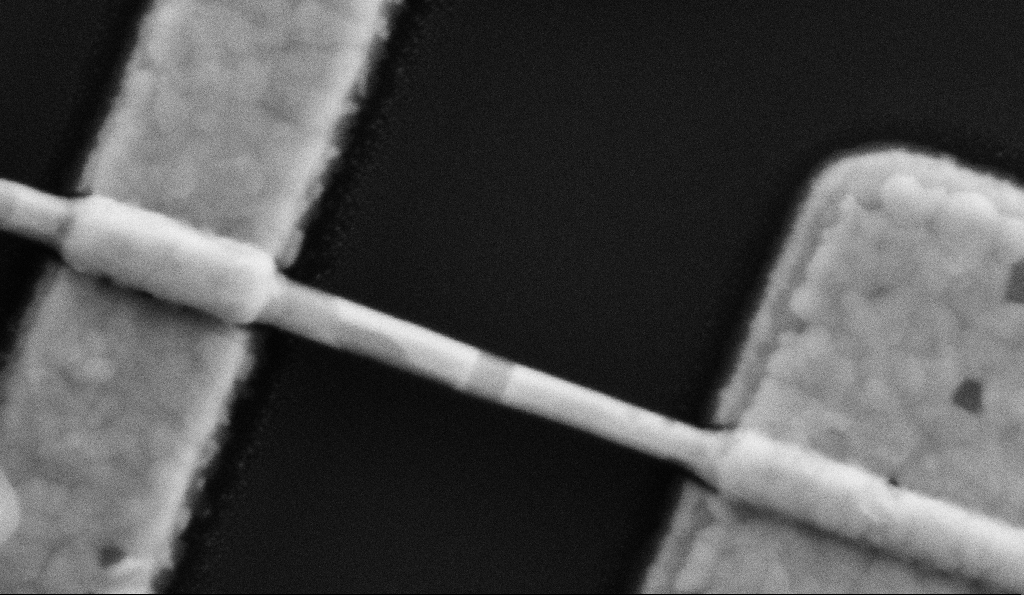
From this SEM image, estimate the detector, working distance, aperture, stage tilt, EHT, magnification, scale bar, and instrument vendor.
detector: SE2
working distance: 8.5 mm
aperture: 30 µm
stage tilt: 45°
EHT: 5 kV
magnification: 200 K X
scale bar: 200 nm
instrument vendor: Zeiss